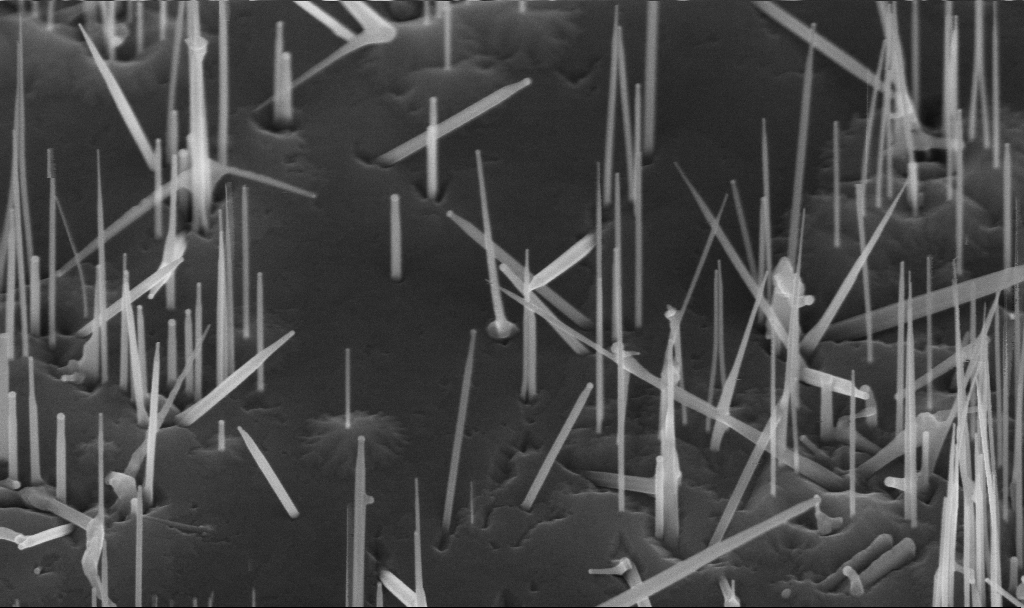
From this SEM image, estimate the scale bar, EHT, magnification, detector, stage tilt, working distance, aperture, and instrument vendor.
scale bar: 200 nm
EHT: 10 kV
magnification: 84.97 K X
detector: InLens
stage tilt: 45°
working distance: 5.6 mm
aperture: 30 µm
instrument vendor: Zeiss